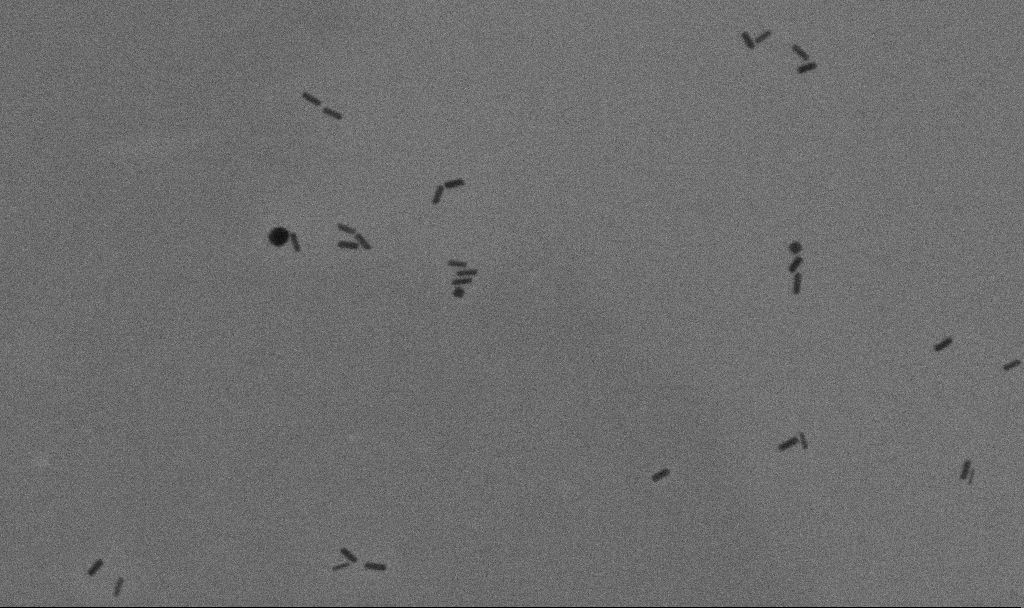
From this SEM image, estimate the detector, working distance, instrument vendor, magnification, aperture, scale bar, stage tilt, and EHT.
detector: SE2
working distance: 11.3 mm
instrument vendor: Zeiss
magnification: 100 K X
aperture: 30 µm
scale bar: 200 nm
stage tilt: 0°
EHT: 10 kV